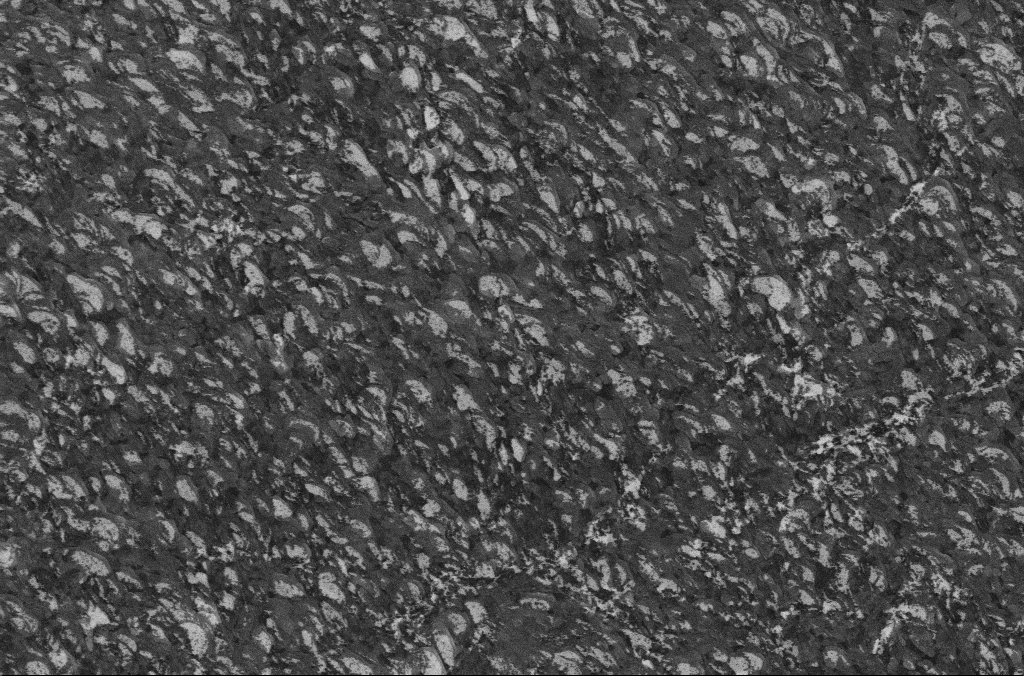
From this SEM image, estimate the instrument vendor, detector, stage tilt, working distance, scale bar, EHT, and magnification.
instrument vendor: Zeiss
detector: InLens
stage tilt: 0°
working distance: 6.7 mm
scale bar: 2000 nm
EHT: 5 kV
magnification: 10 K X